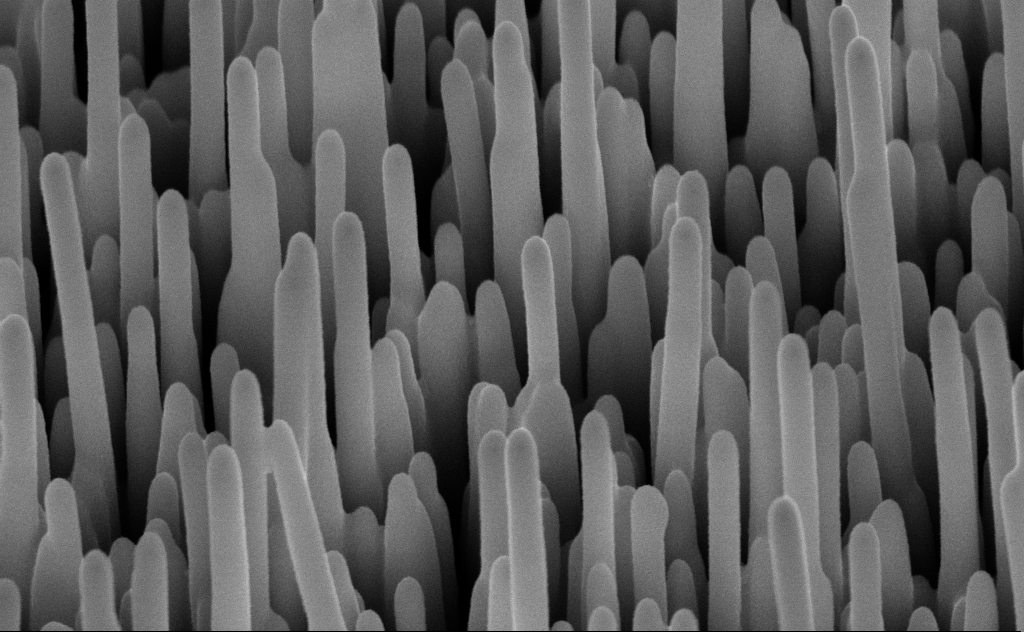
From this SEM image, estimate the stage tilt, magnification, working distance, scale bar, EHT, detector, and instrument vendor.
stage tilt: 45°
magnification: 80 K X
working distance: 8 mm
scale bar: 200 nm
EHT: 10 kV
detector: InLens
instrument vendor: Zeiss